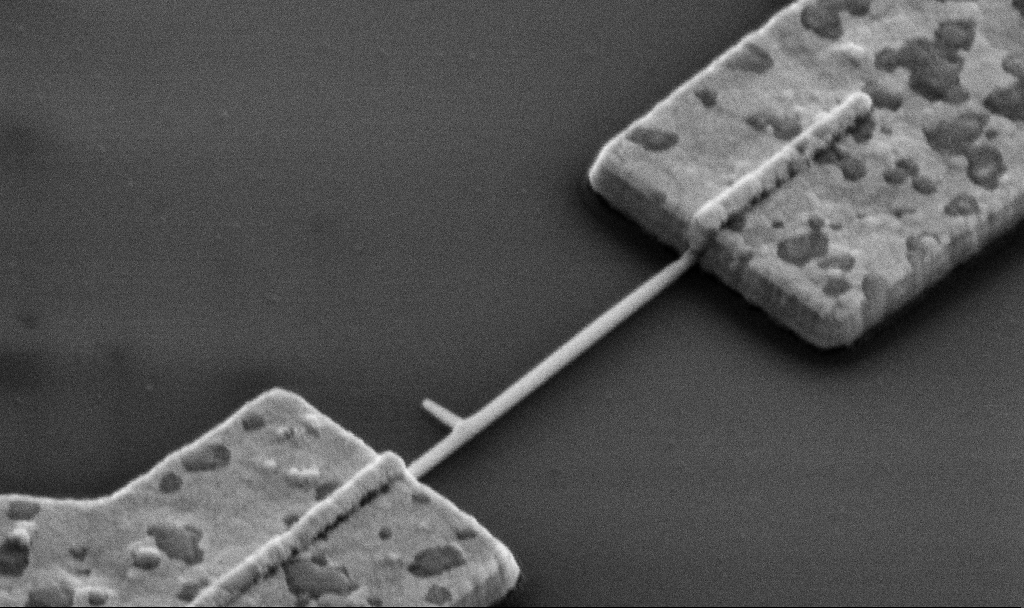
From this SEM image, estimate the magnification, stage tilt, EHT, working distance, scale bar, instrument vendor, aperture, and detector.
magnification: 60 K X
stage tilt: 45°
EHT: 5 kV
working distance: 13.6 mm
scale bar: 1000 nm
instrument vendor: Zeiss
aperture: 30 µm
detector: SE2